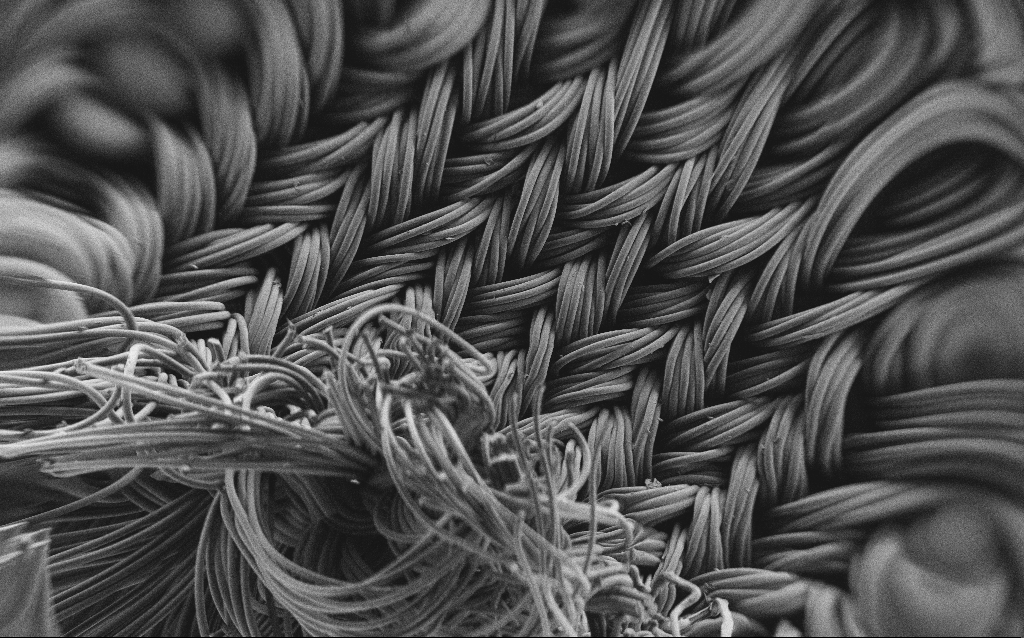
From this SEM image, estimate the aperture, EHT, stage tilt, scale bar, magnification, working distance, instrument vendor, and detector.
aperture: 30 µm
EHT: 1 kV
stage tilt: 0°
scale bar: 100000 nm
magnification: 0.121 K X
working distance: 4 mm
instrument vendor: Zeiss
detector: SE2